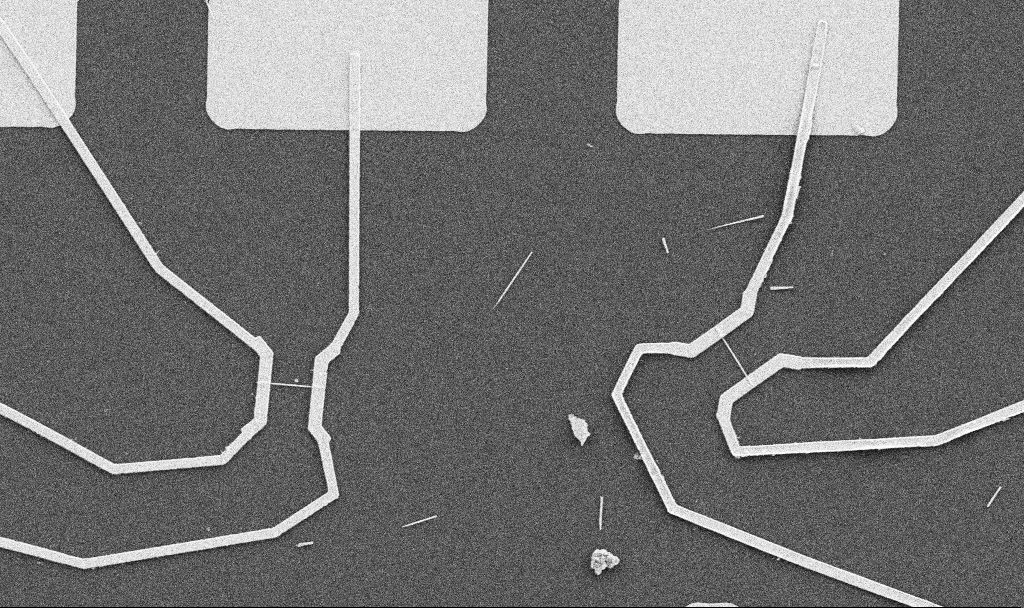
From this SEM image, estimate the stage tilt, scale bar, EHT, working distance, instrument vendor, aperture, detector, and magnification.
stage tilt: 0°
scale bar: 10000 nm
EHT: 5 kV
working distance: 10.7 mm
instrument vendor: Zeiss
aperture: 30 µm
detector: SE2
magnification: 5 K X